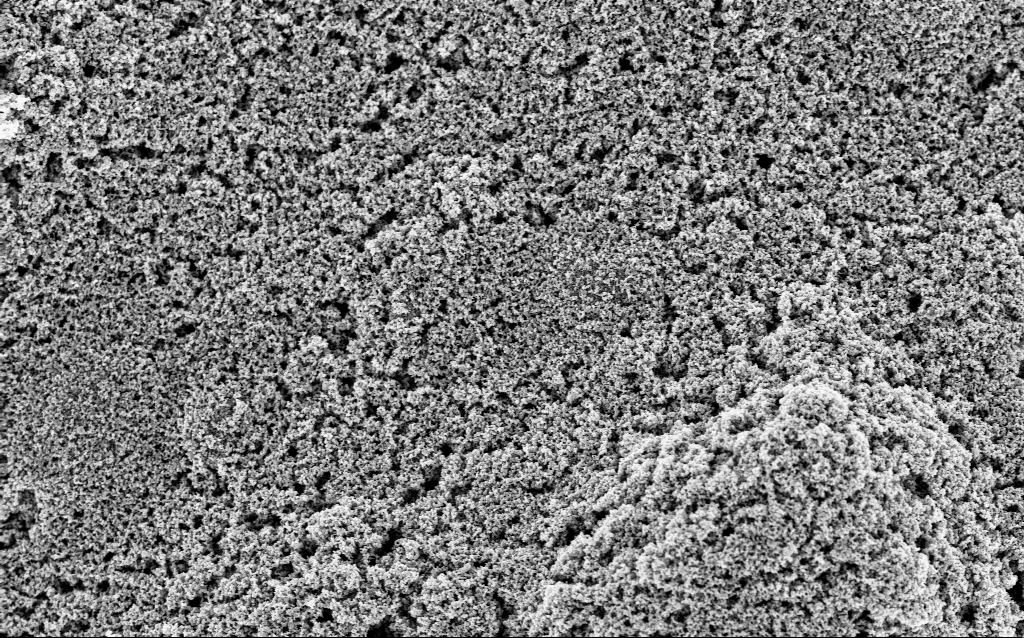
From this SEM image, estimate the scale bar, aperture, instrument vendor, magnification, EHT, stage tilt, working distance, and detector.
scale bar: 1000 nm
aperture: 30 µm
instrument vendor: Zeiss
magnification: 23.9 K X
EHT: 3 kV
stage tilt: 0°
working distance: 7.5 mm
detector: InLens